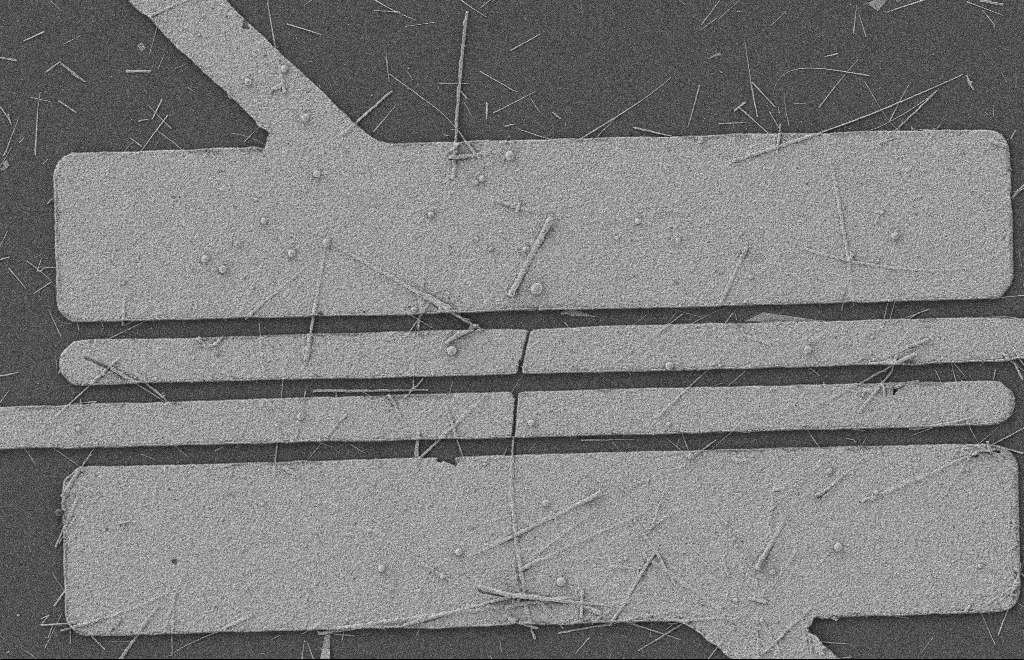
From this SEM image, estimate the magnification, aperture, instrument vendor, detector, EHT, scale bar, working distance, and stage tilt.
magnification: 5.75 K X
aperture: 20 µm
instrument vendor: Zeiss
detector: SE2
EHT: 2 kV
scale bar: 2000 nm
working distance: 8 mm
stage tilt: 0°